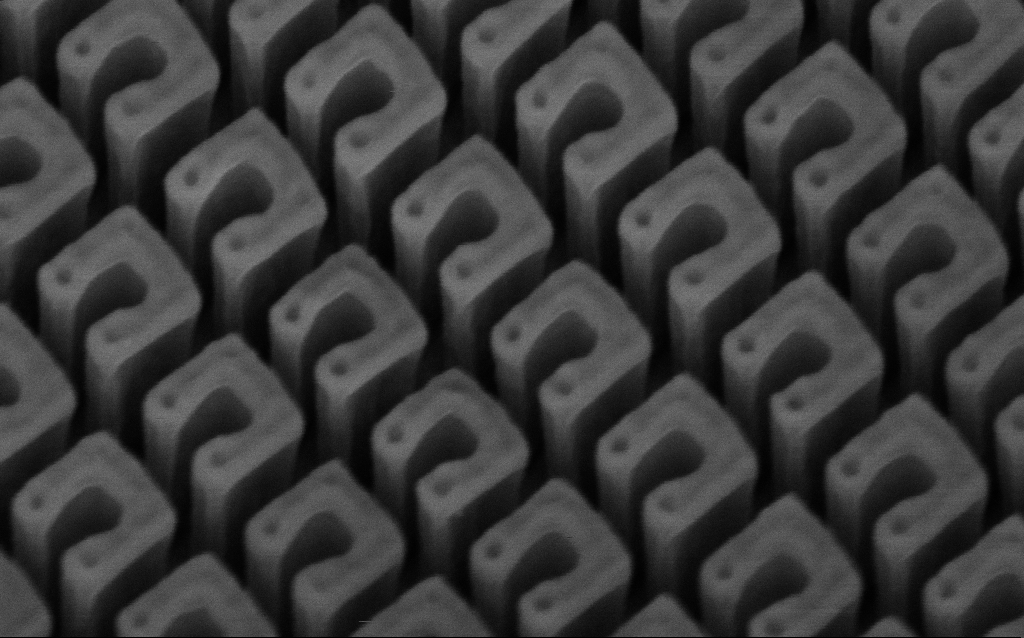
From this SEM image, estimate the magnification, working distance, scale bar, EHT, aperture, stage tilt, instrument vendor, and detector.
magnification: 130.14 K X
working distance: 6.9 mm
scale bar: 200 nm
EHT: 3 kV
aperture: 30 µm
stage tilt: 45°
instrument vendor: Zeiss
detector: InLens